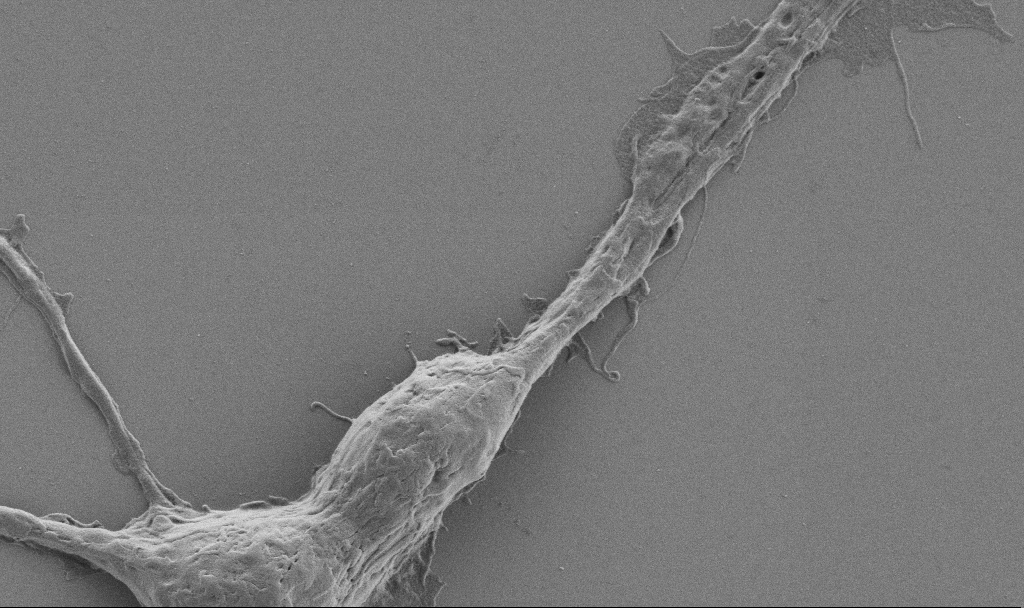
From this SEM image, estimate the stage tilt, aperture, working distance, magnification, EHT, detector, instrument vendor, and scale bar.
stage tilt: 0°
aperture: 30 µm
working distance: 6.9 mm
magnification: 10 K X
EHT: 0.9 kV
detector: SE2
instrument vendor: Zeiss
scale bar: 2000 nm